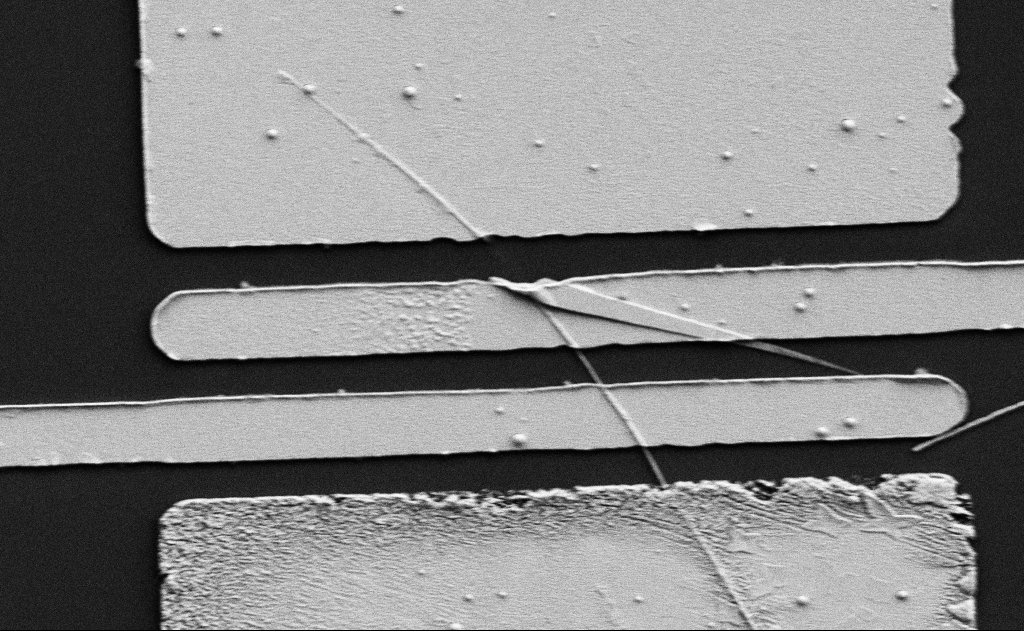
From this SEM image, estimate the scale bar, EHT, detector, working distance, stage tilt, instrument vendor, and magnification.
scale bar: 2000 nm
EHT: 5 kV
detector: SE2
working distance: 15 mm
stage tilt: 0°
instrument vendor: Zeiss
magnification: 10 K X